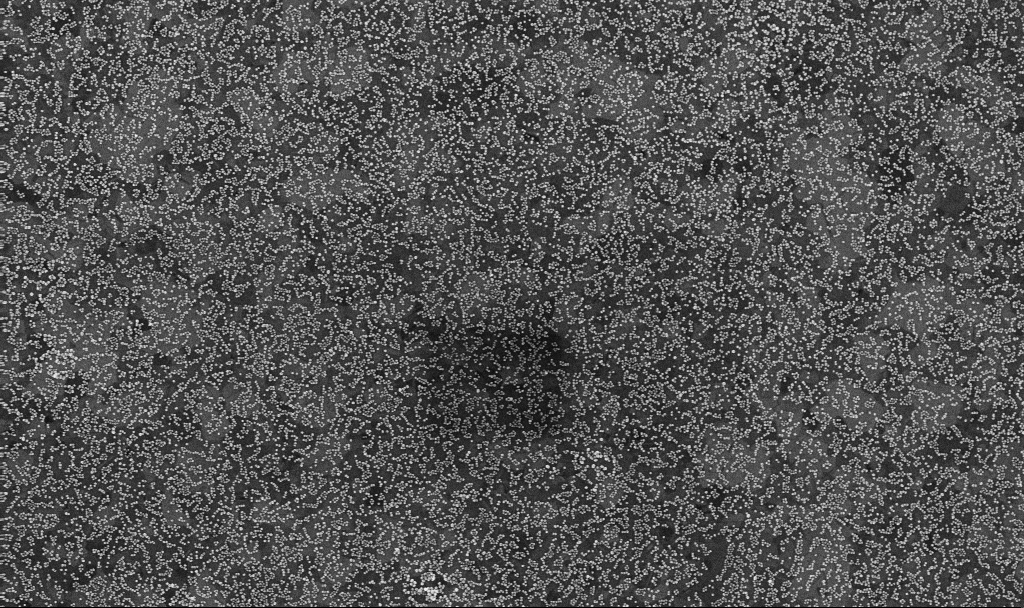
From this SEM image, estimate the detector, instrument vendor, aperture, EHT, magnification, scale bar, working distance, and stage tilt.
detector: InLens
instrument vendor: Zeiss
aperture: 30 µm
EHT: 10 kV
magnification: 50 K X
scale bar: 1000 nm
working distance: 3.3 mm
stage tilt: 0°